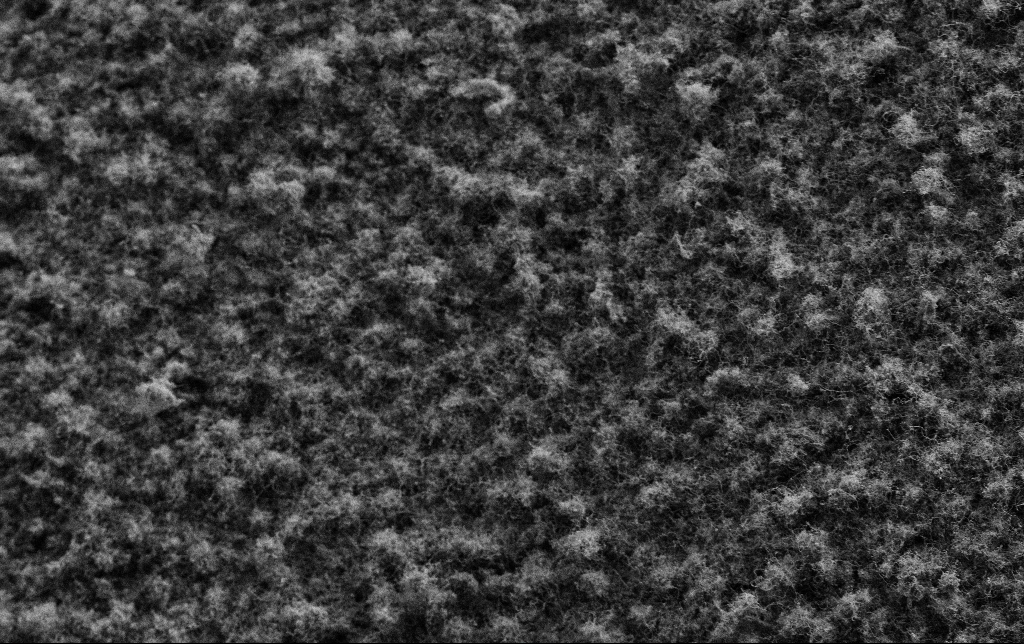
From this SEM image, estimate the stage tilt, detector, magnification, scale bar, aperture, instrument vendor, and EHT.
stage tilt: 0°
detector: SE2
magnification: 2.5 K X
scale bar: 20000 nm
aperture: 30 µm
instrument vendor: Zeiss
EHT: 2 kV